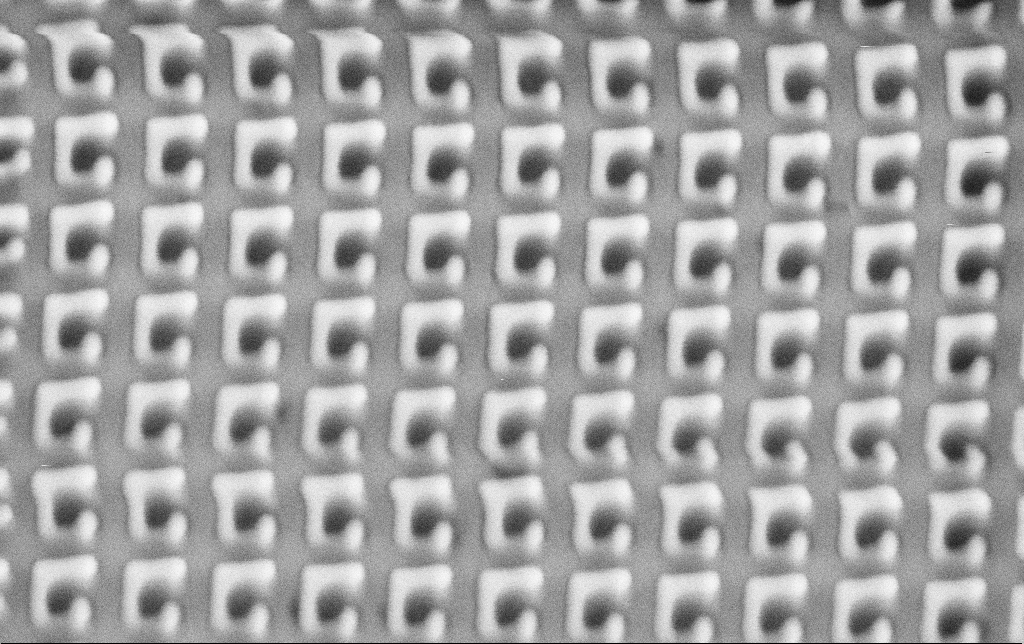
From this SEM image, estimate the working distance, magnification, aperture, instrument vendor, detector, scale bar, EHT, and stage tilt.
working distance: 8.1 mm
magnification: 70.24 K X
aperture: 30 µm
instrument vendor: Zeiss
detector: SE2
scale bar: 1000 nm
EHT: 1.5 kV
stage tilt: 0°